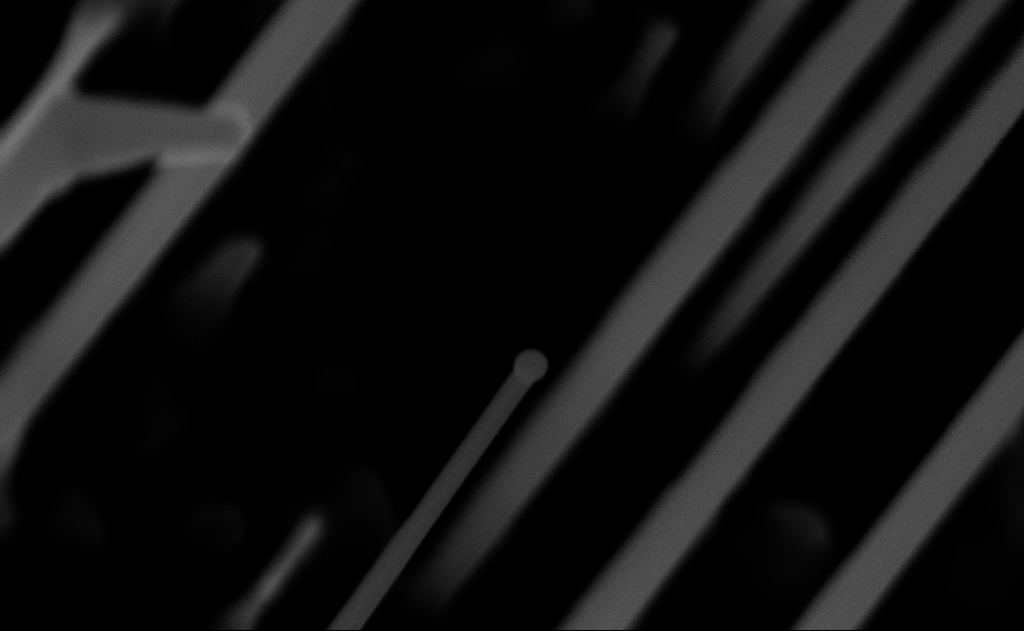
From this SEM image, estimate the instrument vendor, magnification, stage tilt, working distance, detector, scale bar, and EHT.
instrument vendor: Zeiss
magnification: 150 K X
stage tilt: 0°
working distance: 10 mm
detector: InLens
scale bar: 100 nm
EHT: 10 kV